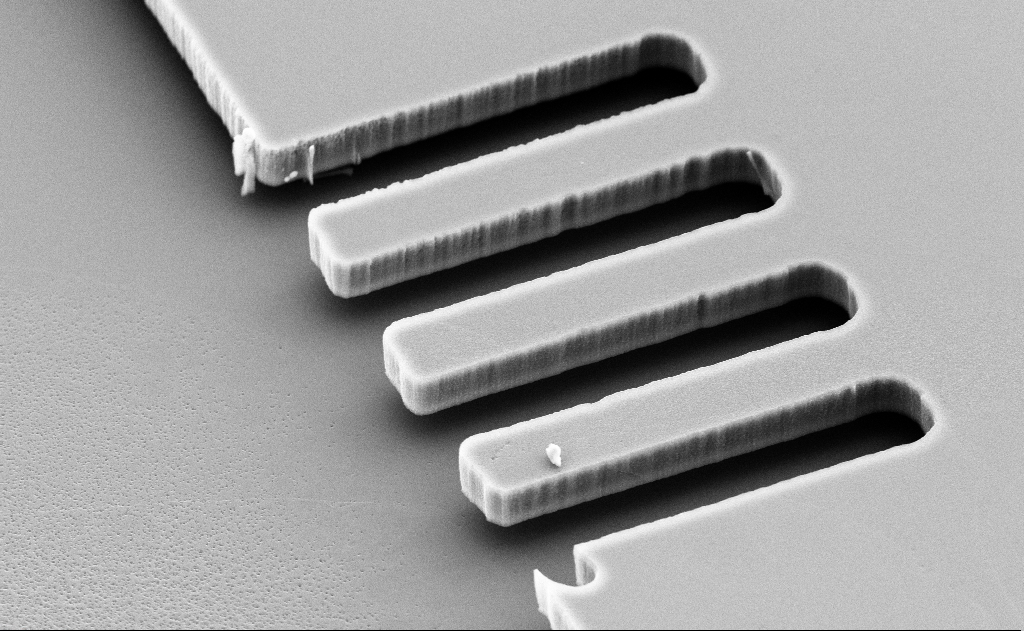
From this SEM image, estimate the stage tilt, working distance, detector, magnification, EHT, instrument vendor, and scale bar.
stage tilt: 46°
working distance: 11 mm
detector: SE2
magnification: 6.7 K X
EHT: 10 kV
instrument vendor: Zeiss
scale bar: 10000 nm